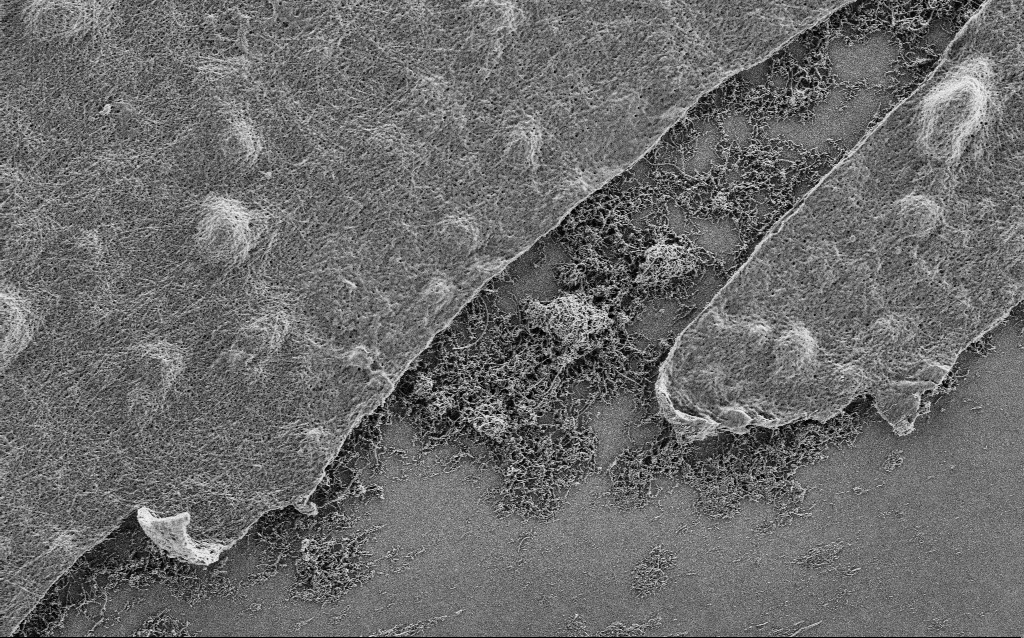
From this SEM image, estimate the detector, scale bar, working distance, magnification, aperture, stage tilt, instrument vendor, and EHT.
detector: SE2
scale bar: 2000 nm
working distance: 4 mm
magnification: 10 K X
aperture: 30 µm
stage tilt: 0°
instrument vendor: Zeiss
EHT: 1 kV